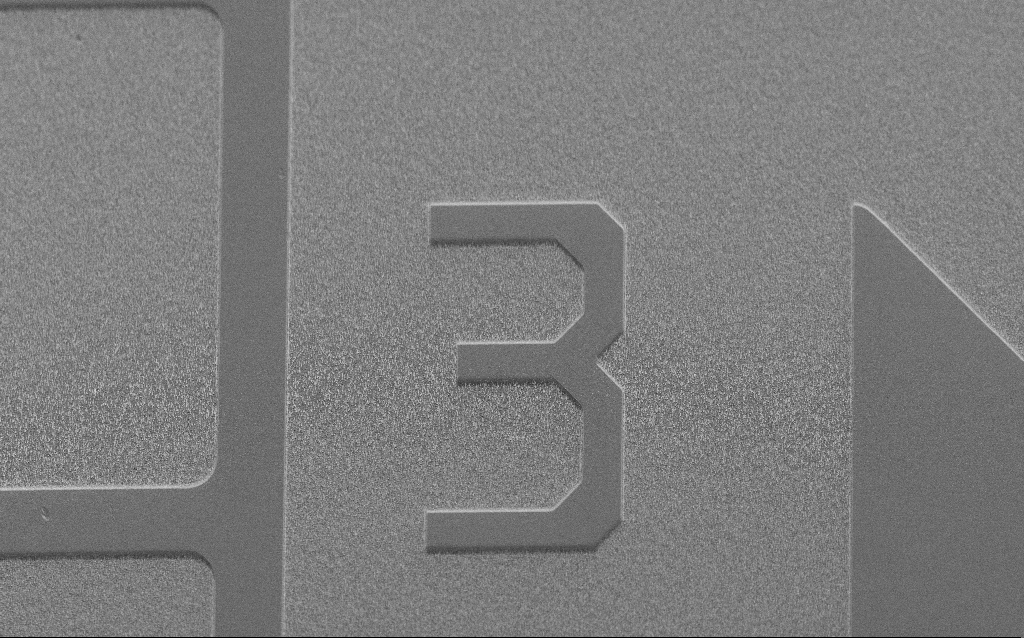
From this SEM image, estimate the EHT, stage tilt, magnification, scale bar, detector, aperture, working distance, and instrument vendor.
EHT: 5 kV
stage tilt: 45°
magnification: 0.521 K X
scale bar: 100000 nm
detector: SE2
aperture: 30 µm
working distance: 8 mm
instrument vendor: Zeiss